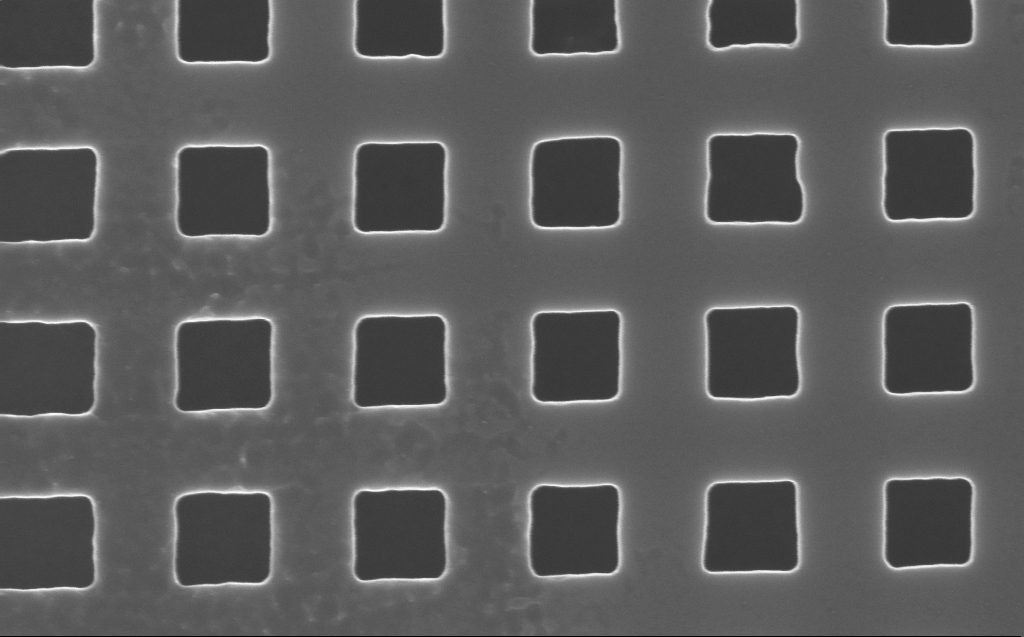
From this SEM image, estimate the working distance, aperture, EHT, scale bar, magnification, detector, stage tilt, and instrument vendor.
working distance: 4 mm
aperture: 30 µm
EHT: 10 kV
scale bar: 200 nm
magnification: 131.08 K X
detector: InLens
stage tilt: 0°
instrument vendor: Zeiss